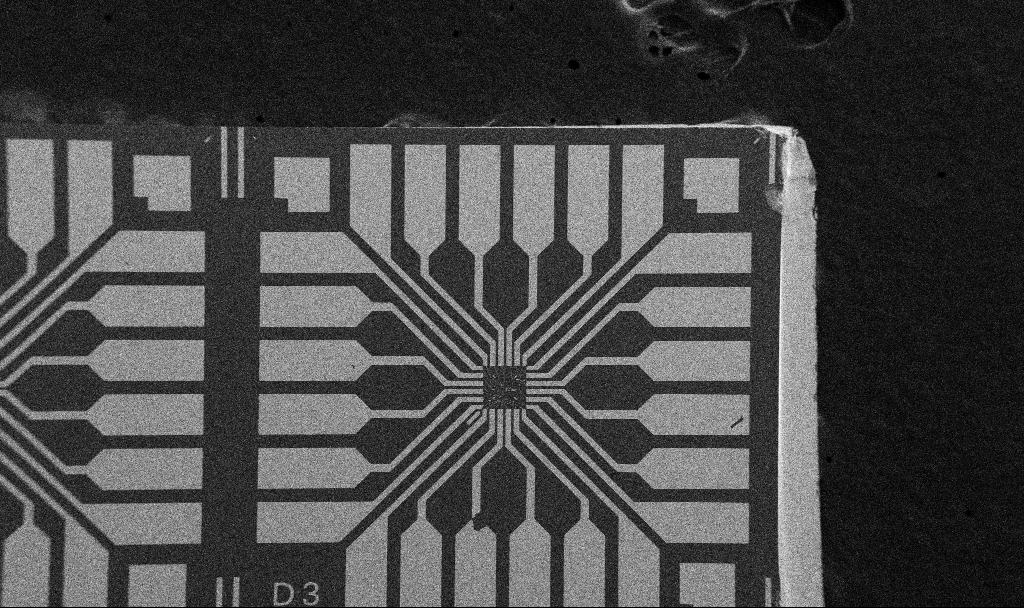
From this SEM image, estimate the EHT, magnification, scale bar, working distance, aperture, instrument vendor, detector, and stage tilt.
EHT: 5 kV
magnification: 0.1 K X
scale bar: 200000 nm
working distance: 10.7 mm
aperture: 30 µm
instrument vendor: Zeiss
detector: SE2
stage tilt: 0°